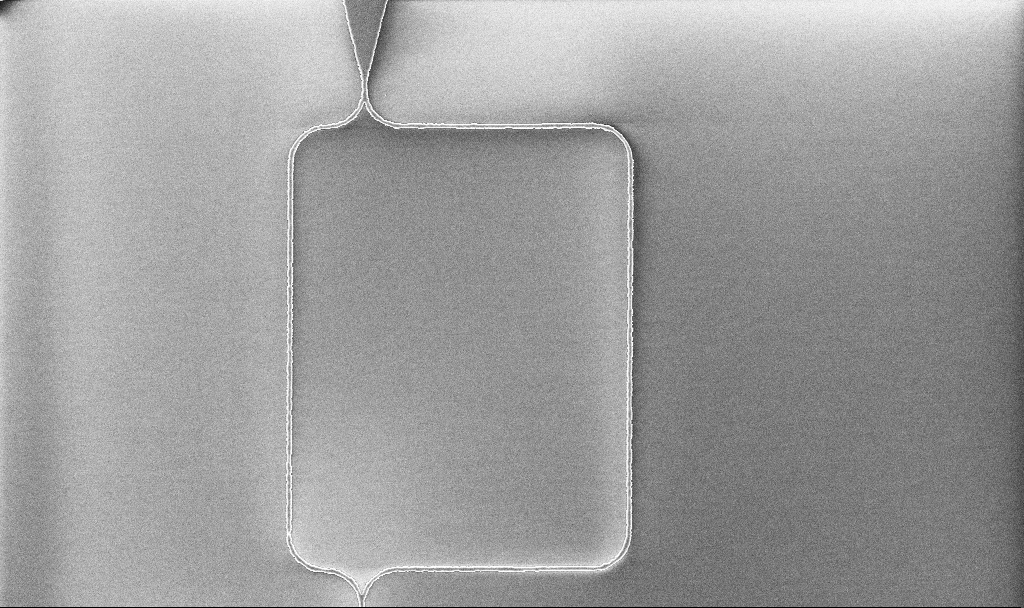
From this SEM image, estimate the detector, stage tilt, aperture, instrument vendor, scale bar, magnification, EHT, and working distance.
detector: InLens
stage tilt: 0°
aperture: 30 µm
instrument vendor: Zeiss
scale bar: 10000 nm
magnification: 2.86 K X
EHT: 5 kV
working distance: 10.1 mm